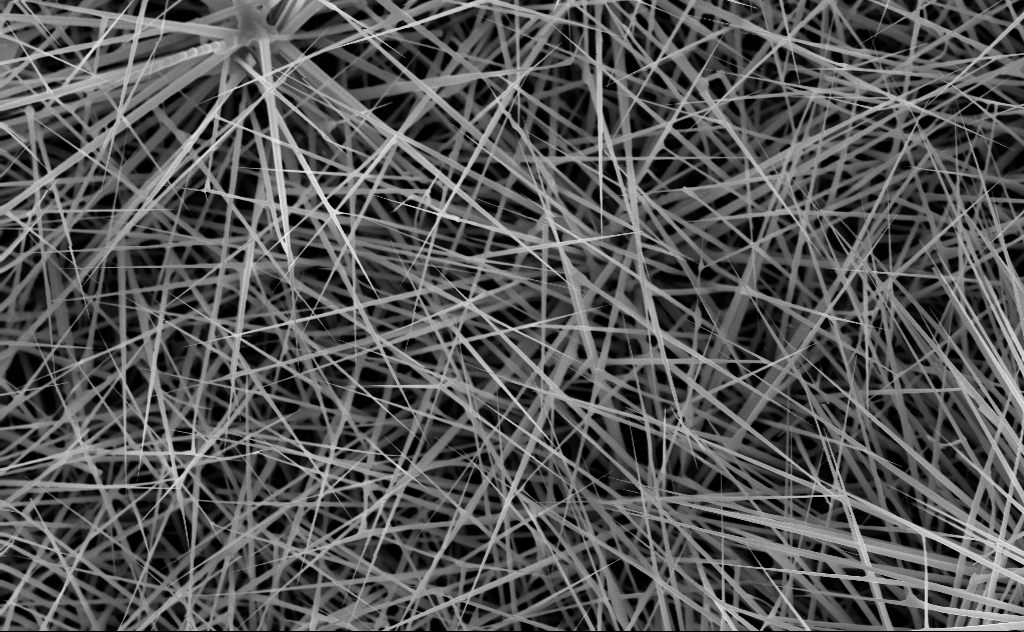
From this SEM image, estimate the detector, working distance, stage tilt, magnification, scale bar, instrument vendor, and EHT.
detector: InLens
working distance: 10 mm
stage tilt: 0°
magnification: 20 K X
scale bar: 2000 nm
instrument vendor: Zeiss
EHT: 10 kV